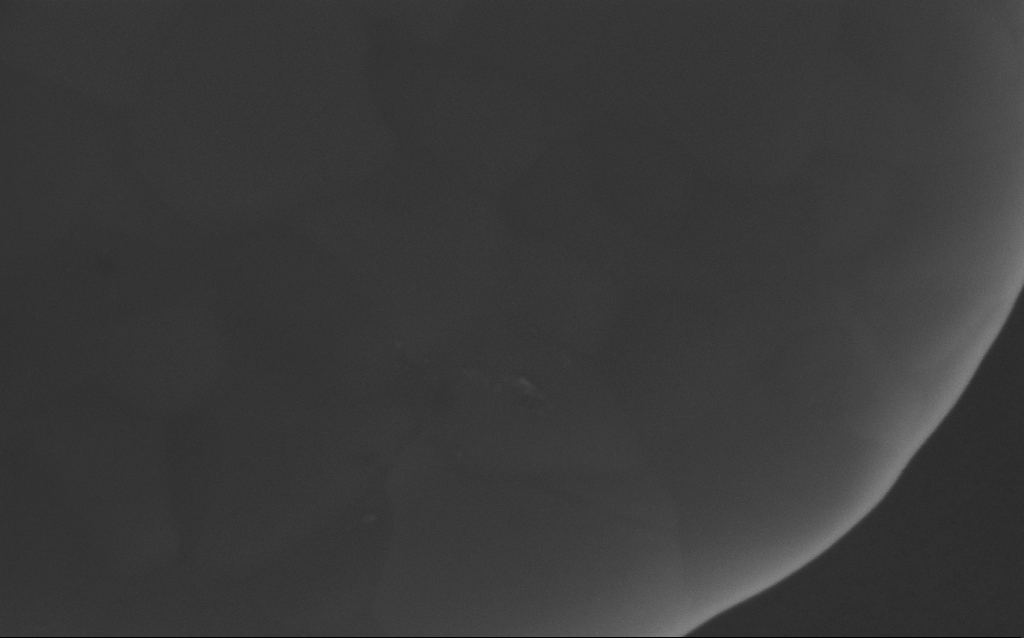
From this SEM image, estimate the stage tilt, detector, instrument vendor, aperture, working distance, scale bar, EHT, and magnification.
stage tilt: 0°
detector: InLens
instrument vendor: Zeiss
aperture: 30 µm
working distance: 3 mm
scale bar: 200 nm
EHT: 5 kV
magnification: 256.52 K X